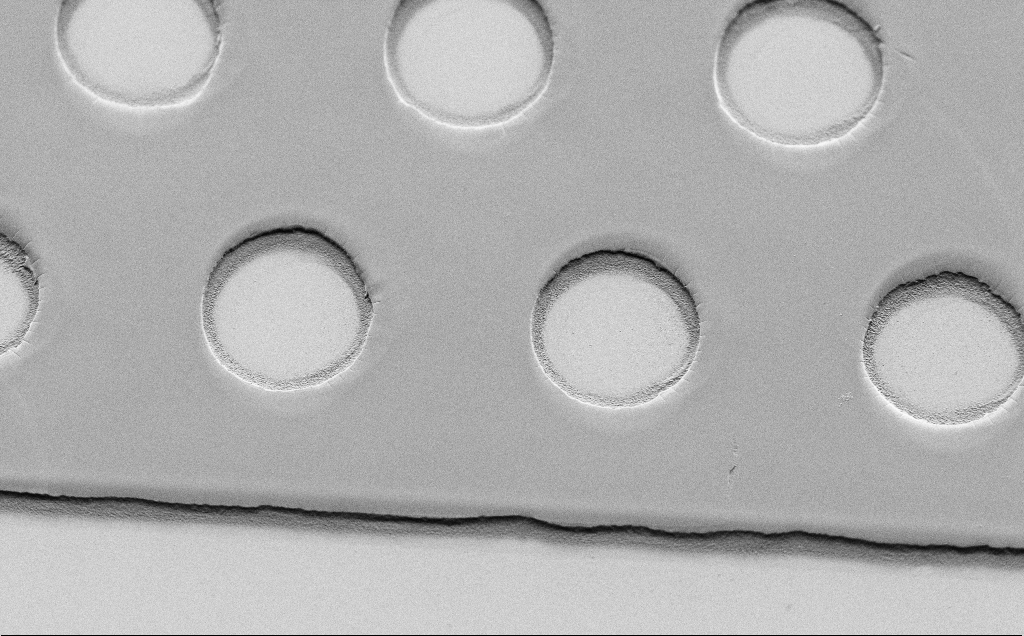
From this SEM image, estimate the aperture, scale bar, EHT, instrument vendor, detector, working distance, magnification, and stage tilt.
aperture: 30 µm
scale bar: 20000 nm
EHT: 1.5 kV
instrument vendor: Zeiss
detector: SE2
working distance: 6 mm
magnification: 2.42 K X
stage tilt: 45°